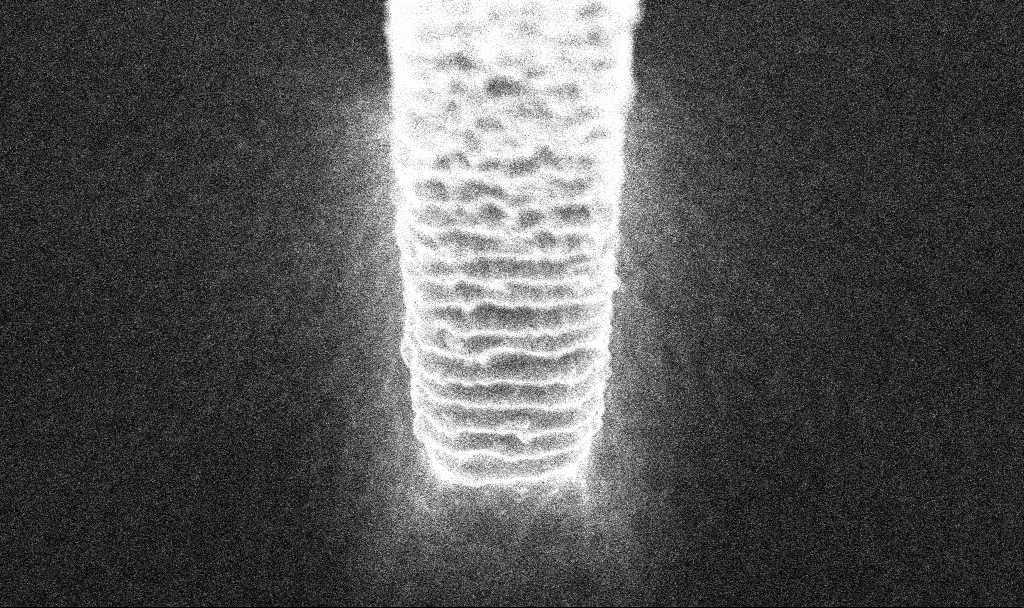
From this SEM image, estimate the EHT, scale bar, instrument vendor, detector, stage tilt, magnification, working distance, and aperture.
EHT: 5 kV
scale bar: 1000 nm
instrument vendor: Zeiss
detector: InLens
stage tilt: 20°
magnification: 56.42 K X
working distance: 4.5 mm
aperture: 30 µm